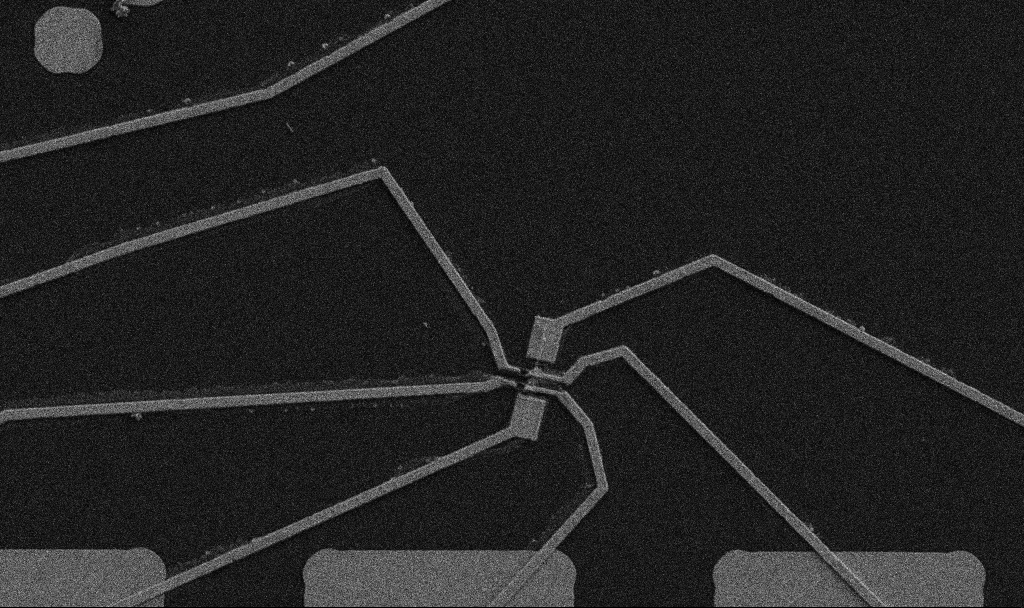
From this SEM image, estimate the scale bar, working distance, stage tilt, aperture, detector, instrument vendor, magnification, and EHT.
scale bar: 10000 nm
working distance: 10.7 mm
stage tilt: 0°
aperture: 30 µm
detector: SE2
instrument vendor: Zeiss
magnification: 5 K X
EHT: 5 kV